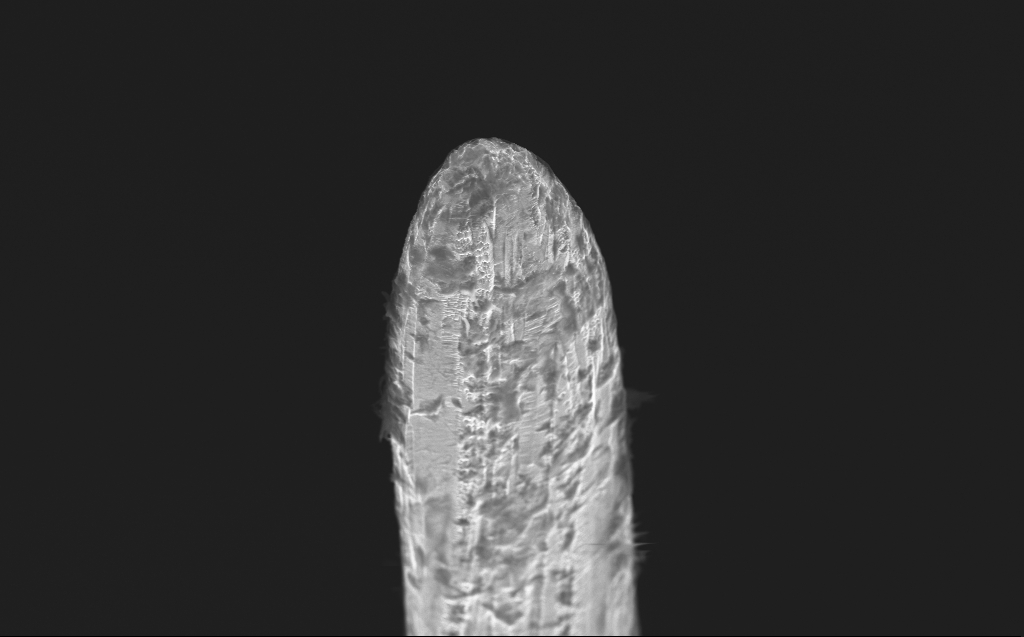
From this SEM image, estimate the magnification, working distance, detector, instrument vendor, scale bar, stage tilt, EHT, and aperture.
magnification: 11.33 K X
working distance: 4 mm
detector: InLens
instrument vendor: Zeiss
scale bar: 2000 nm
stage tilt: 40°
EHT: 10 kV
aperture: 30 µm